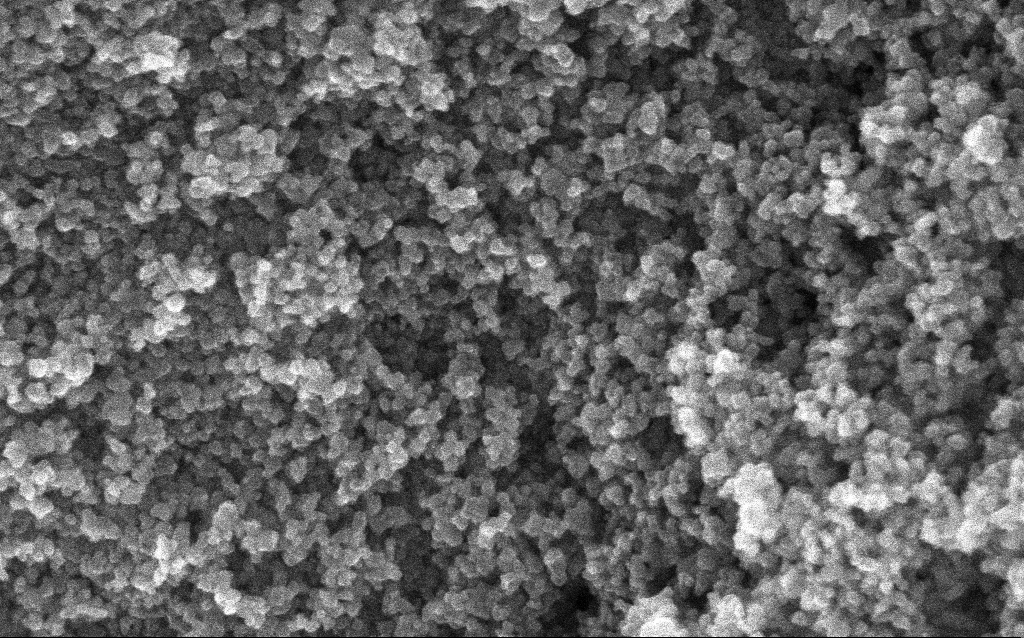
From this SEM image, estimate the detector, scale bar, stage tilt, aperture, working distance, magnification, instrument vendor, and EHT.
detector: InLens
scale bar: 100 nm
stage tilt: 0°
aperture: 30 µm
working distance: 2.6 mm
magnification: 204.13 K X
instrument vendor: Zeiss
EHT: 10 kV